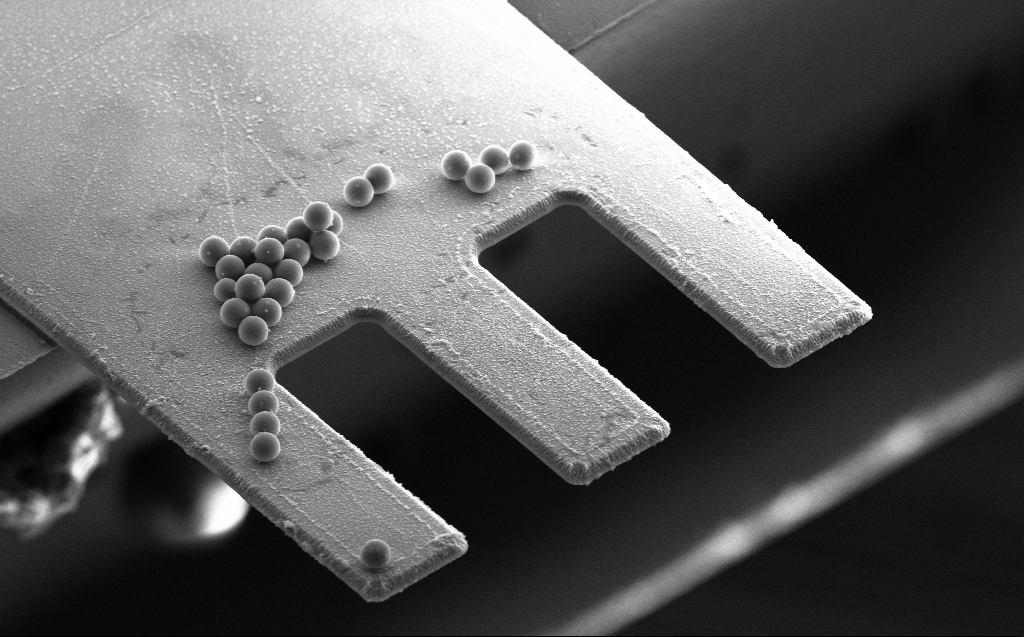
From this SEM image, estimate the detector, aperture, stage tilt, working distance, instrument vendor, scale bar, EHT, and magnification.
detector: SE2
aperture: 30 µm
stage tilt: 45.4°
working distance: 11 mm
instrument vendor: Zeiss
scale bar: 20000 nm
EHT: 10 kV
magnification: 2.4 K X